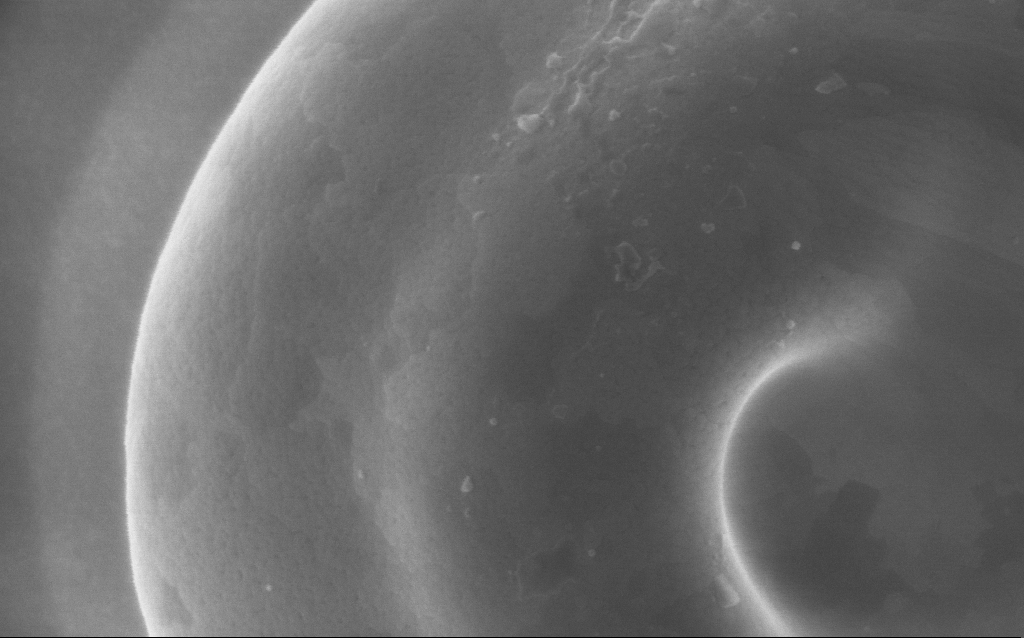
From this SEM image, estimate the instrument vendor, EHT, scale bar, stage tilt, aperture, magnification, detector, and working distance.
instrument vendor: Zeiss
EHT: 5 kV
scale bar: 200 nm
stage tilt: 0°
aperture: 30 µm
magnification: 136 K X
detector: InLens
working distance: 4 mm